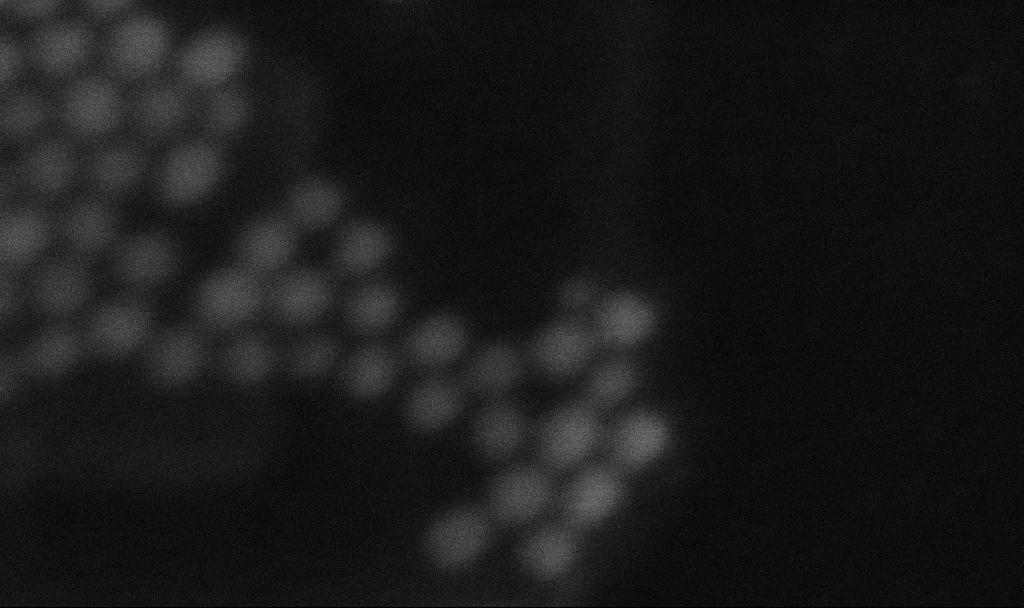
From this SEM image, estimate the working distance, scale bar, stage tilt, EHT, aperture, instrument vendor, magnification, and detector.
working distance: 3.3 mm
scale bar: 20 nm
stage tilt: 0°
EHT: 10 kV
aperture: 30 µm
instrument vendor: Zeiss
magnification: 1179.41 K X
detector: InLens